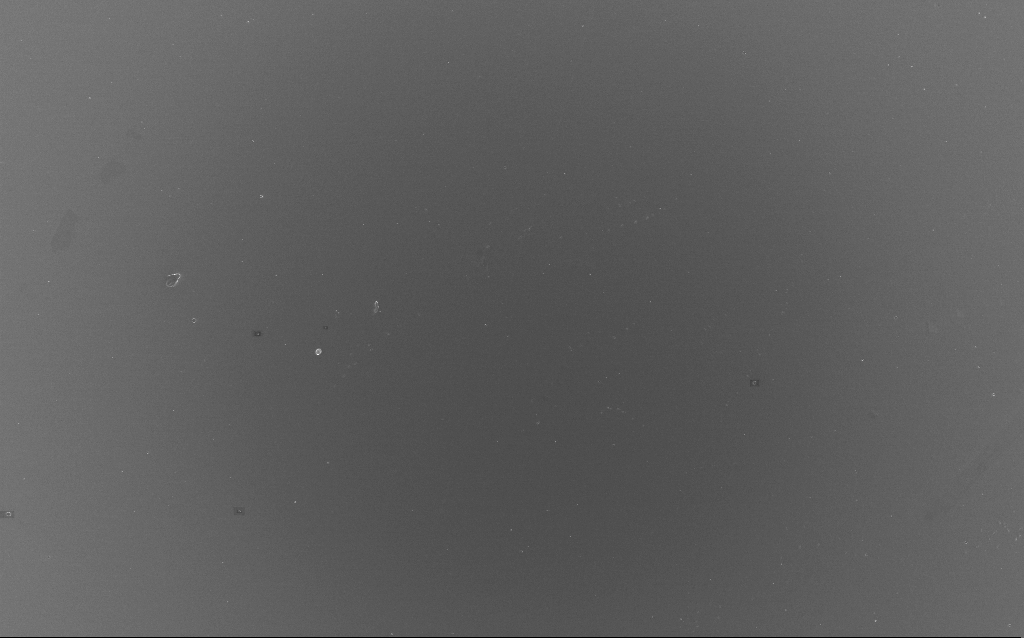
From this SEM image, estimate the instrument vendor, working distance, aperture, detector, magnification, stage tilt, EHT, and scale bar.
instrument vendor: Zeiss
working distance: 2 mm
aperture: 30 µm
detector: InLens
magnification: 0.599 K X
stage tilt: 0°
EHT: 10 kV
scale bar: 100000 nm